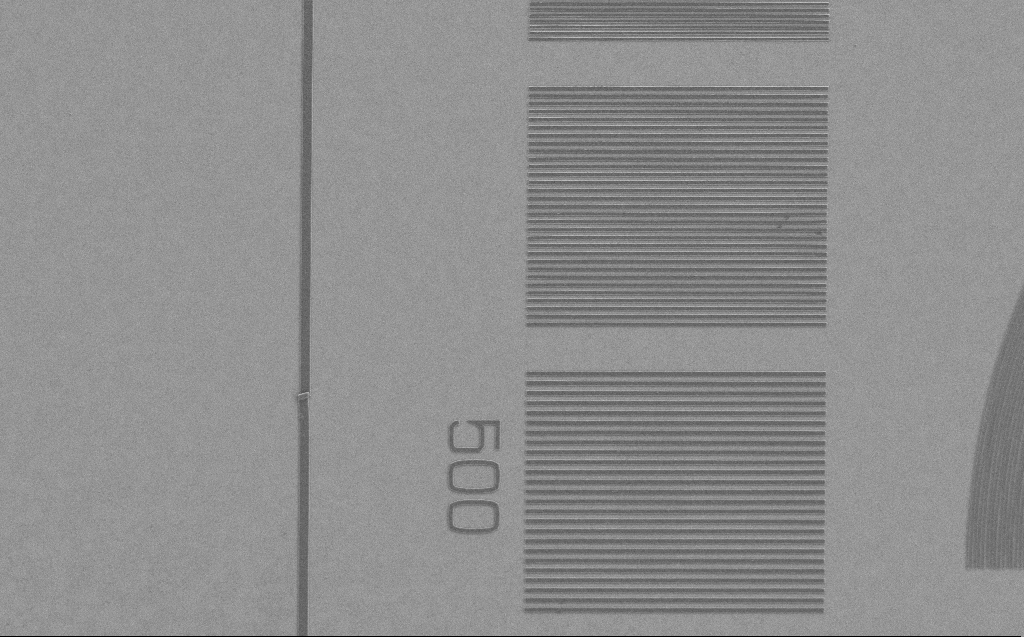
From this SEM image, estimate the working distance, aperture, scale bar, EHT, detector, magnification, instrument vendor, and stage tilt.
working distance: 5 mm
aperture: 30 µm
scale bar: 10000 nm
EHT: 1.2 kV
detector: SE2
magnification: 3.65 K X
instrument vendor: Zeiss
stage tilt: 0°